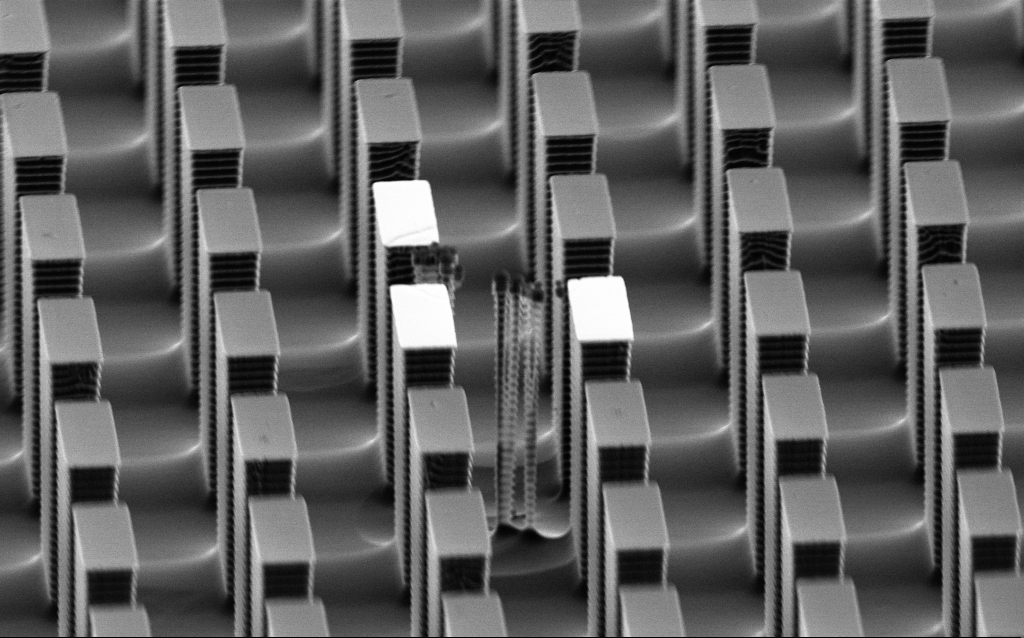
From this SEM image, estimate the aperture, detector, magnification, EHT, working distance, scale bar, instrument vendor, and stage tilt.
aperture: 30 µm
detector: SE2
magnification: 11.54 K X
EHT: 3 kV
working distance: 10 mm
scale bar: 2000 nm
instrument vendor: Zeiss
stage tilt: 67°